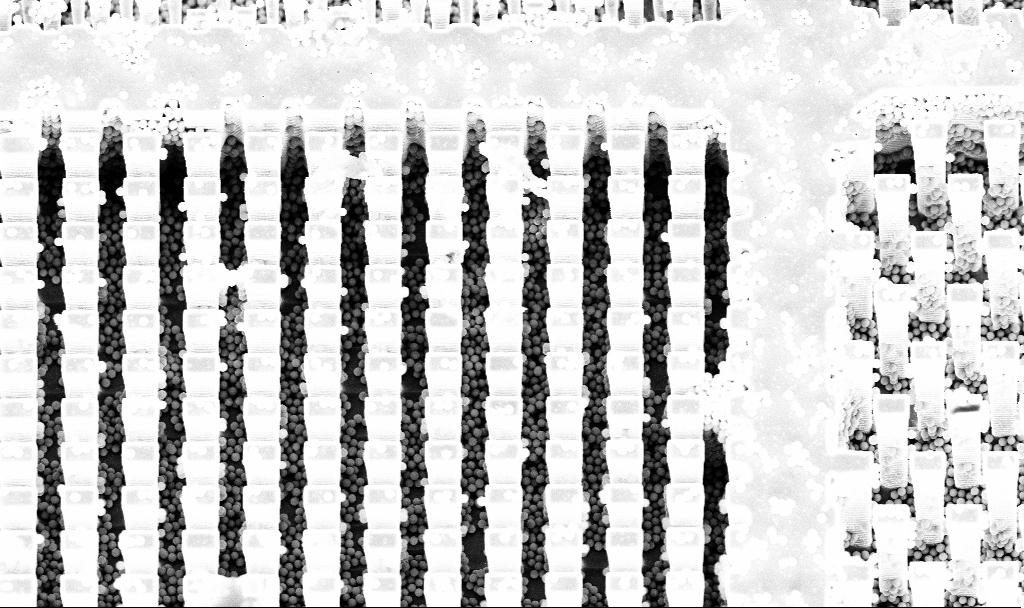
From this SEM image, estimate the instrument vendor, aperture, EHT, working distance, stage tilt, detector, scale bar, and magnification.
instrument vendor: Zeiss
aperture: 30 µm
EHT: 5 kV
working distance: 4.6 mm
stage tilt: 20°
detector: InLens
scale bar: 10000 nm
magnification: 5.49 K X